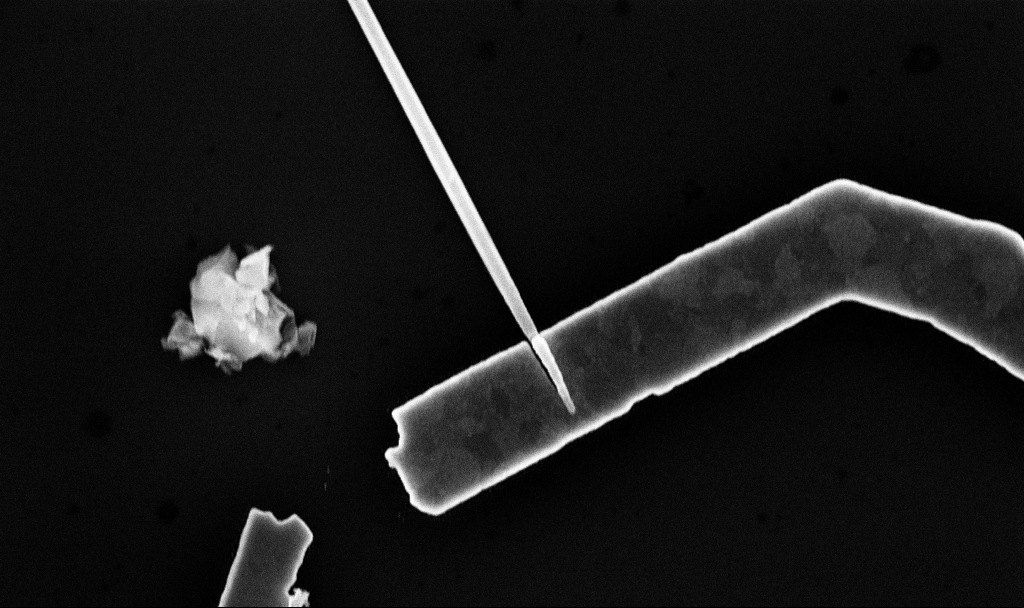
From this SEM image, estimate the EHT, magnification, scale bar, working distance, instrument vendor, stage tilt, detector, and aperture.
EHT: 10 kV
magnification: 44.67 K X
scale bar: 1000 nm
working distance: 7.7 mm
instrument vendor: Zeiss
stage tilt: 0°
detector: InLens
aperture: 30 µm